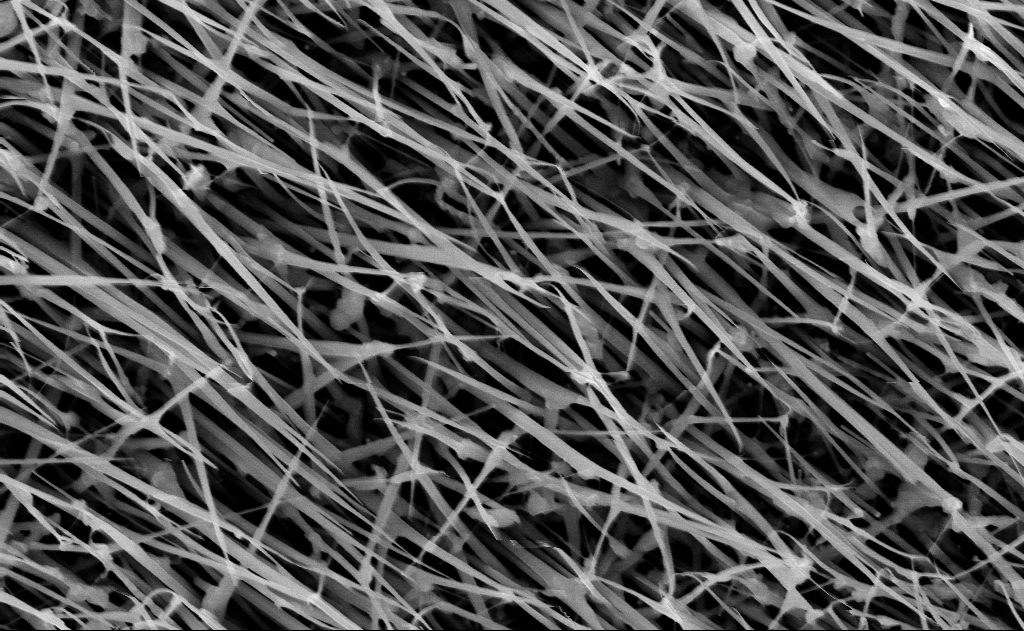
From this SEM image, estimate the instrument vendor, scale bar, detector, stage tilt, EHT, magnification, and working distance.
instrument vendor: Zeiss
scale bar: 1000 nm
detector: InLens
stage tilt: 0°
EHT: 10 kV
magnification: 40 K X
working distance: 13 mm